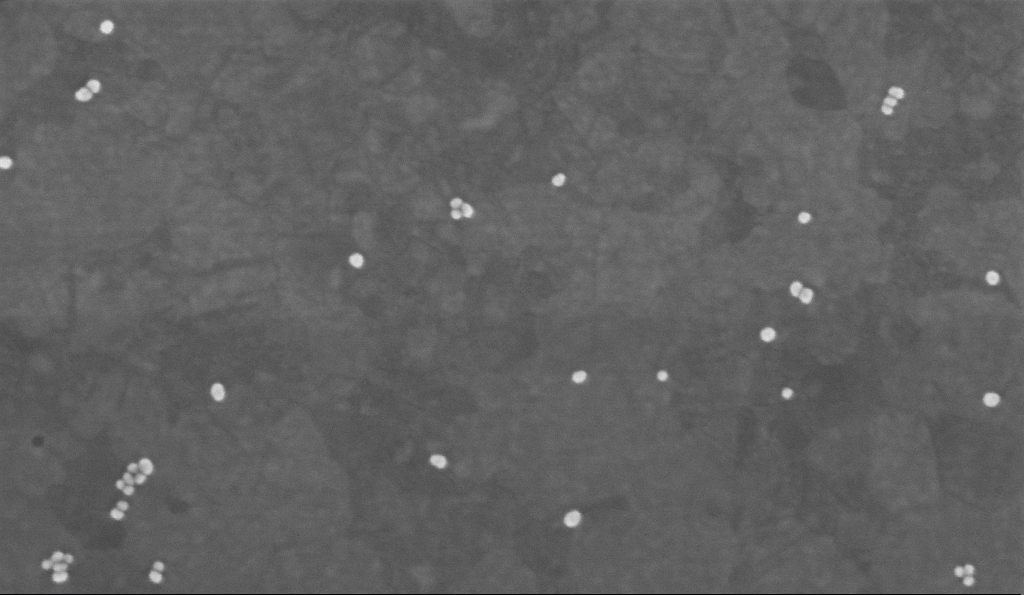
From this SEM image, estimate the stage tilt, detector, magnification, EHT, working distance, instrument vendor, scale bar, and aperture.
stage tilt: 0°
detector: InLens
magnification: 200 K X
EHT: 2 kV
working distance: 3.4 mm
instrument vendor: Zeiss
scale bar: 200 nm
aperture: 30 µm